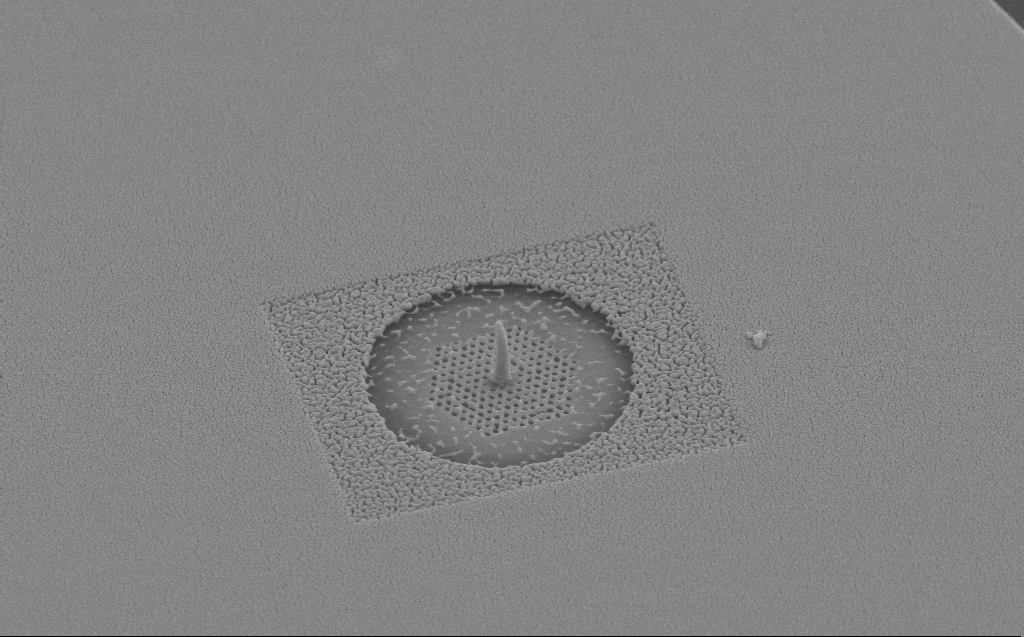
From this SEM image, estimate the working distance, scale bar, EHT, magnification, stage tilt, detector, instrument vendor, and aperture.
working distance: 6 mm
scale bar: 2000 nm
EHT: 10 kV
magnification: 13.87 K X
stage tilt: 45°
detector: SE2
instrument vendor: Zeiss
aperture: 30 µm